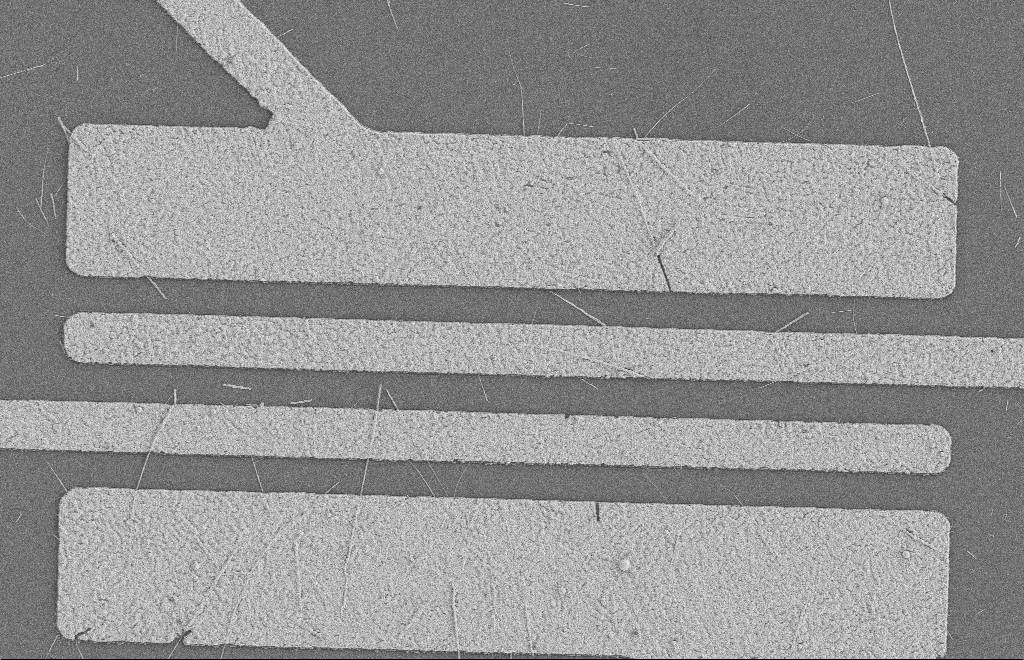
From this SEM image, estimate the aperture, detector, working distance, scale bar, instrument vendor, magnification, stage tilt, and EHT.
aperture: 20 µm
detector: SE2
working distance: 8 mm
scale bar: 2000 nm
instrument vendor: Zeiss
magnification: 5.37 K X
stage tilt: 0°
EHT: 2 kV